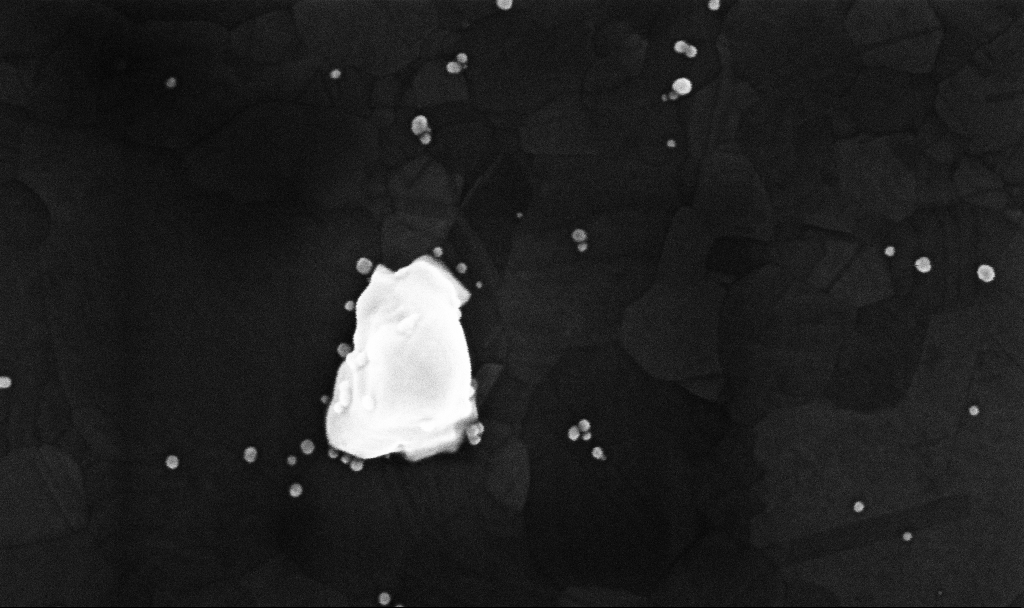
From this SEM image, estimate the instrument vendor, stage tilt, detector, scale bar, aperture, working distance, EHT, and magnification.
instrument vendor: Zeiss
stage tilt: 0°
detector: InLens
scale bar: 200 nm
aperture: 30 µm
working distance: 3.4 mm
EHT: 10 kV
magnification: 229.63 K X